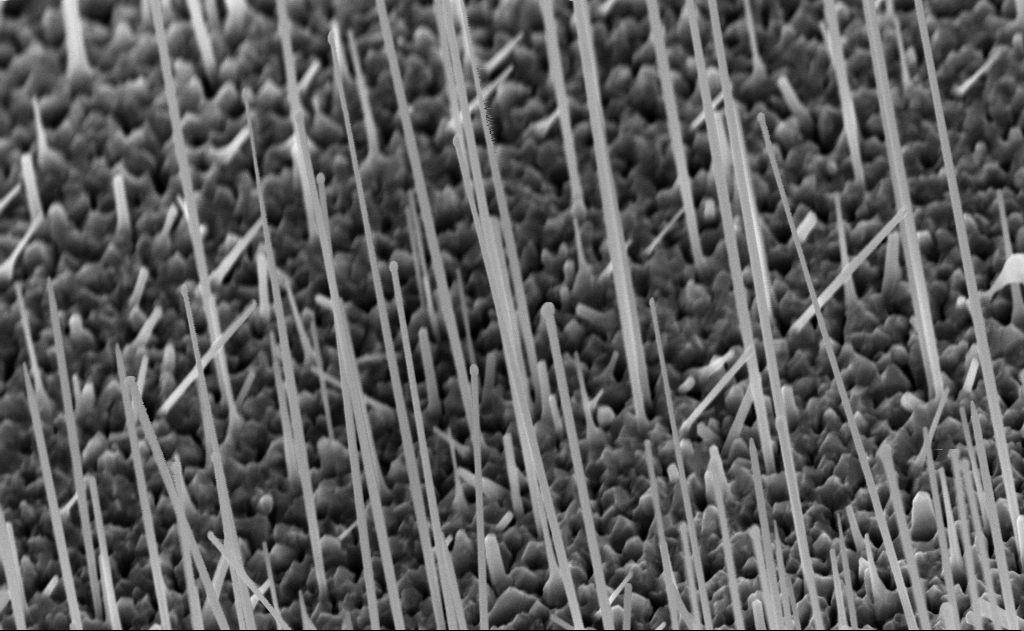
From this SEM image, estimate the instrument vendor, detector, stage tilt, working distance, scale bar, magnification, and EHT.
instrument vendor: Zeiss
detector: InLens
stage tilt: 0°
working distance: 8 mm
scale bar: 1000 nm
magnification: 40 K X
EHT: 10 kV